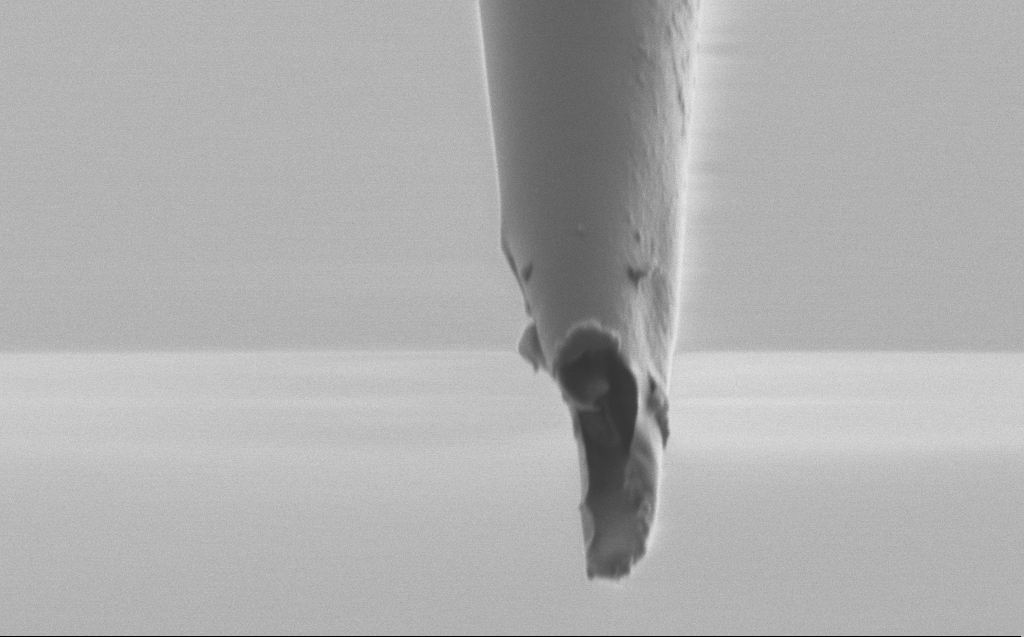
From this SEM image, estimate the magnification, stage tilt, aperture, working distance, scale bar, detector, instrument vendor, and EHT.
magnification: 50 K X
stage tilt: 45°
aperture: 30 µm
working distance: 5 mm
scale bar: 1000 nm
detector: SE2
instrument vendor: Zeiss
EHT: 1 kV